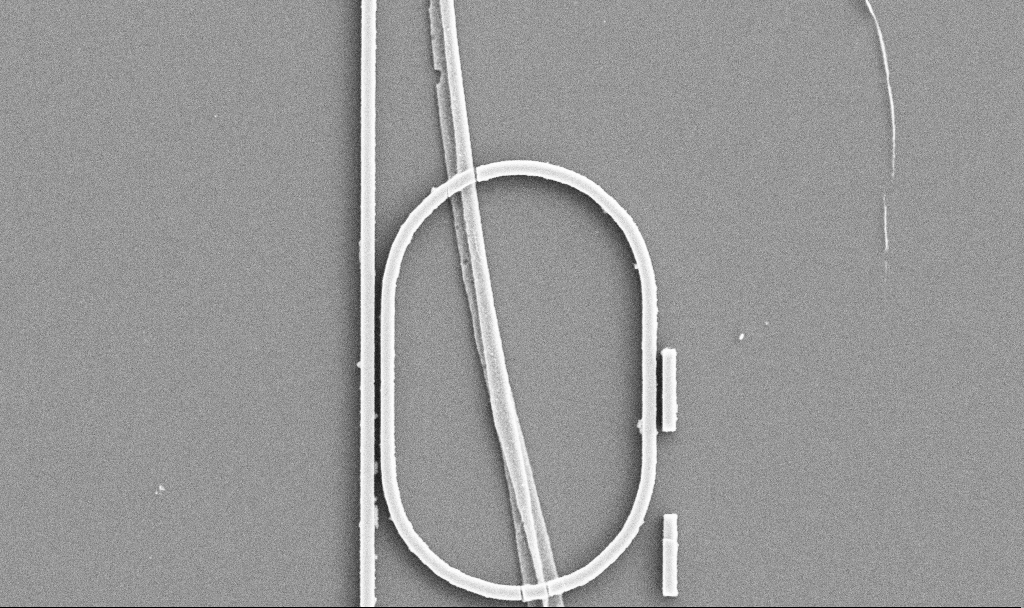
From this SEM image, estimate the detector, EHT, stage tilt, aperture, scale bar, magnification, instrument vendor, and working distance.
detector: SE2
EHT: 5 kV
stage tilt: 0°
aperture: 30 µm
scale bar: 2000 nm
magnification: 10.15 K X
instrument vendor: Zeiss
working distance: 7.4 mm